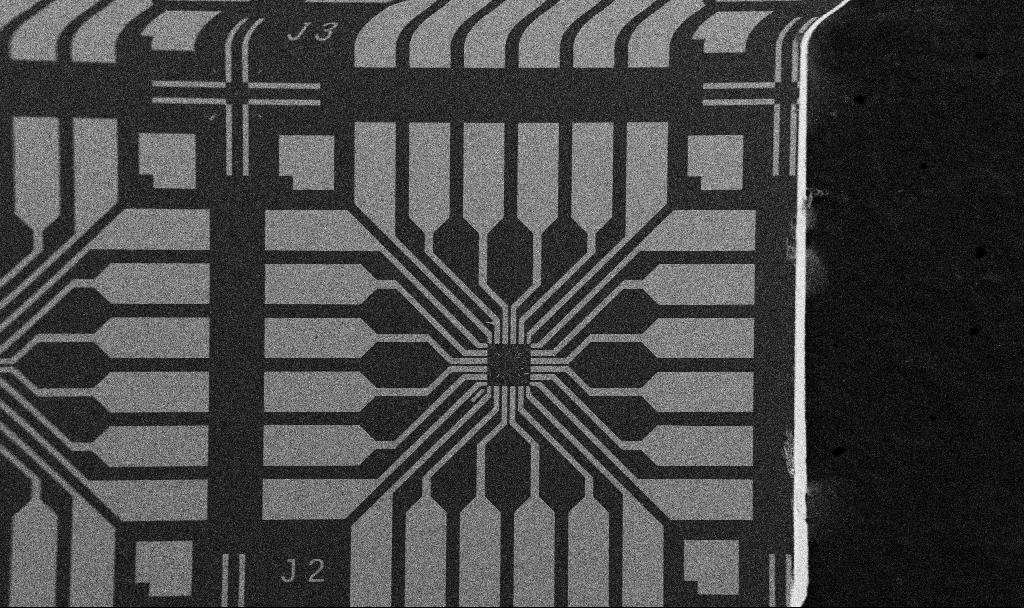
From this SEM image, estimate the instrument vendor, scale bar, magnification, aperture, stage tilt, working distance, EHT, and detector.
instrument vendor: Zeiss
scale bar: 200000 nm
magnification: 0.1 K X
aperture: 30 µm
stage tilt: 0°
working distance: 10.7 mm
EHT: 5 kV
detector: SE2